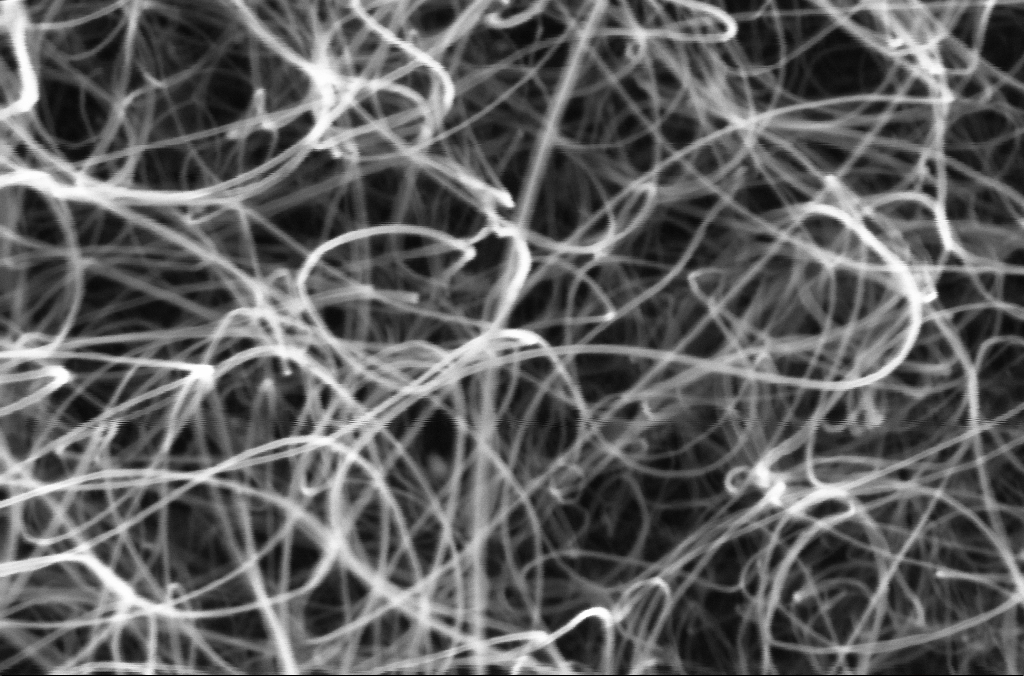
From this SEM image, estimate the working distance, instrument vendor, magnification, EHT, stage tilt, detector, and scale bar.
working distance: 4.4 mm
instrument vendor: Zeiss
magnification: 200 K X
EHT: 5 kV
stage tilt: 0°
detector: InLens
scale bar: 200 nm